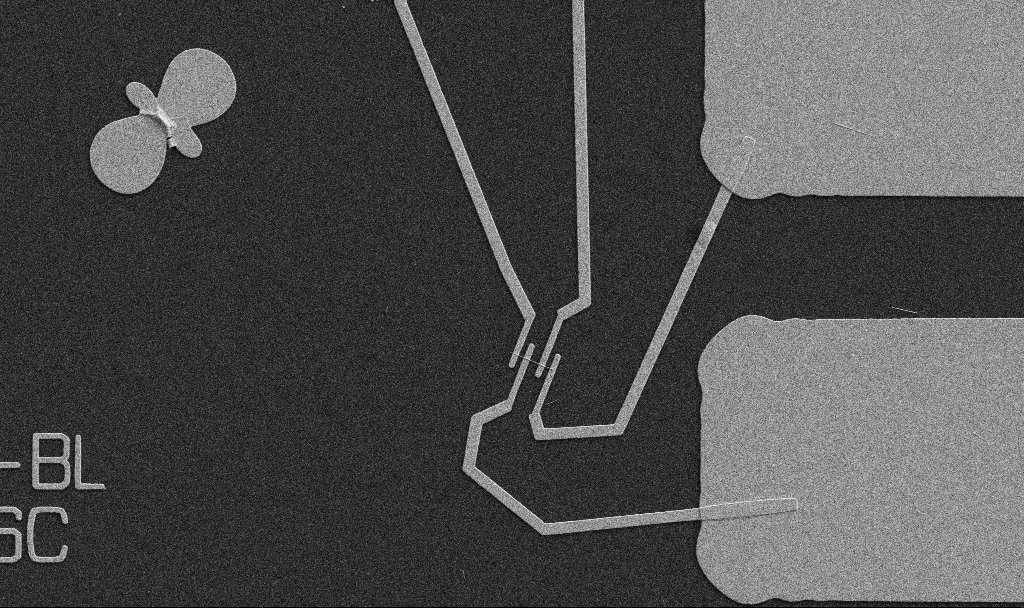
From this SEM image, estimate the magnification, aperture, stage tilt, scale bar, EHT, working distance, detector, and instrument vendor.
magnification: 5 K X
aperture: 30 µm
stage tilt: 0°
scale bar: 10000 nm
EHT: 5 kV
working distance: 10.7 mm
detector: SE2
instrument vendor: Zeiss